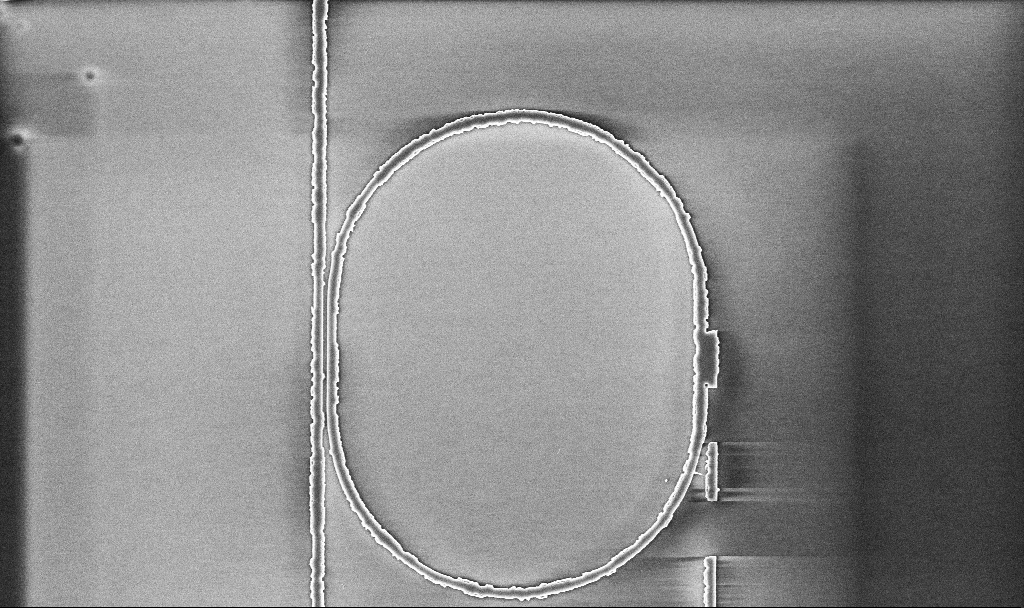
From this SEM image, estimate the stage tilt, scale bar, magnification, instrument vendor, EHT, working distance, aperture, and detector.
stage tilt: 0°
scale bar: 10000 nm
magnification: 6.98 K X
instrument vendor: Zeiss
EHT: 5 kV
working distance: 10.1 mm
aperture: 30 µm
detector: InLens